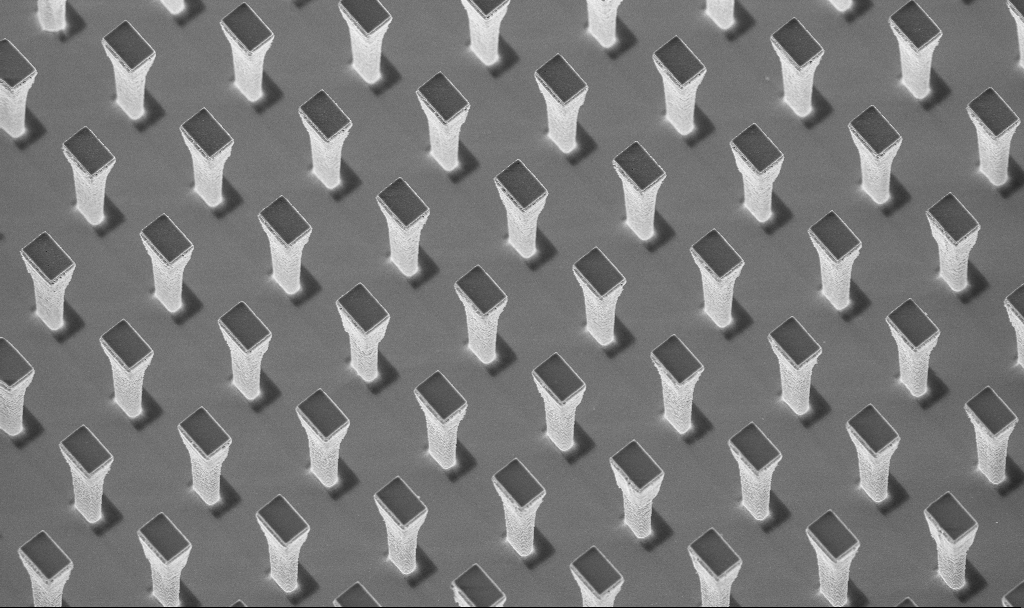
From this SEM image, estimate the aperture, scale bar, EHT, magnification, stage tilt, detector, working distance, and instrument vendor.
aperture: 30 µm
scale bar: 10000 nm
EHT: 5 kV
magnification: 3.75 K X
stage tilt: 20°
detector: InLens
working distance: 4.3 mm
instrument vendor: Zeiss